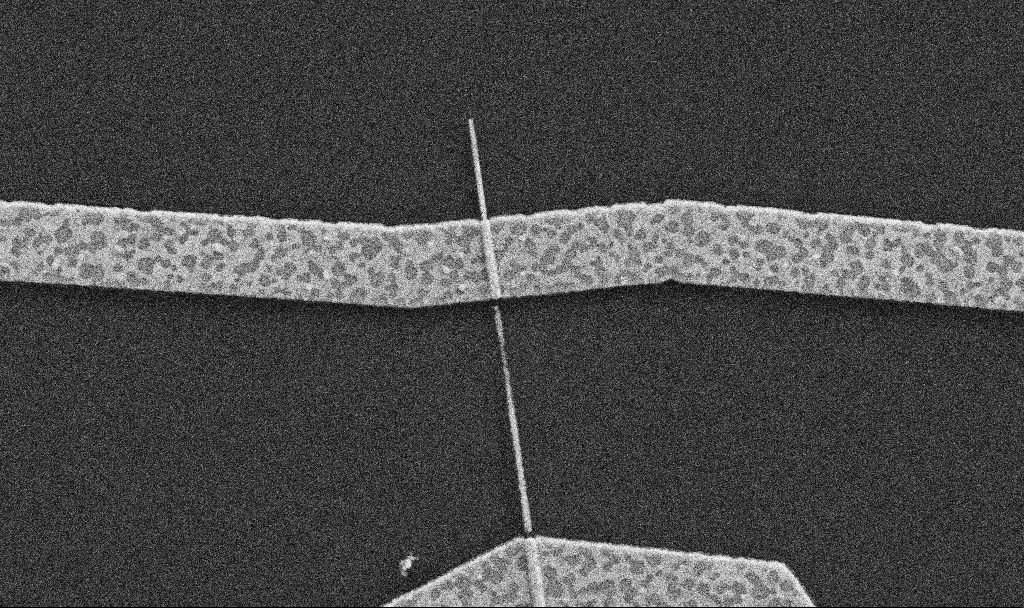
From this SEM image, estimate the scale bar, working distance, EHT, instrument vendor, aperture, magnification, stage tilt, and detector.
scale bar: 1000 nm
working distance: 8.7 mm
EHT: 5 kV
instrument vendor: Zeiss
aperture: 30 µm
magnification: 30 K X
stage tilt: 0°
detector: SE2